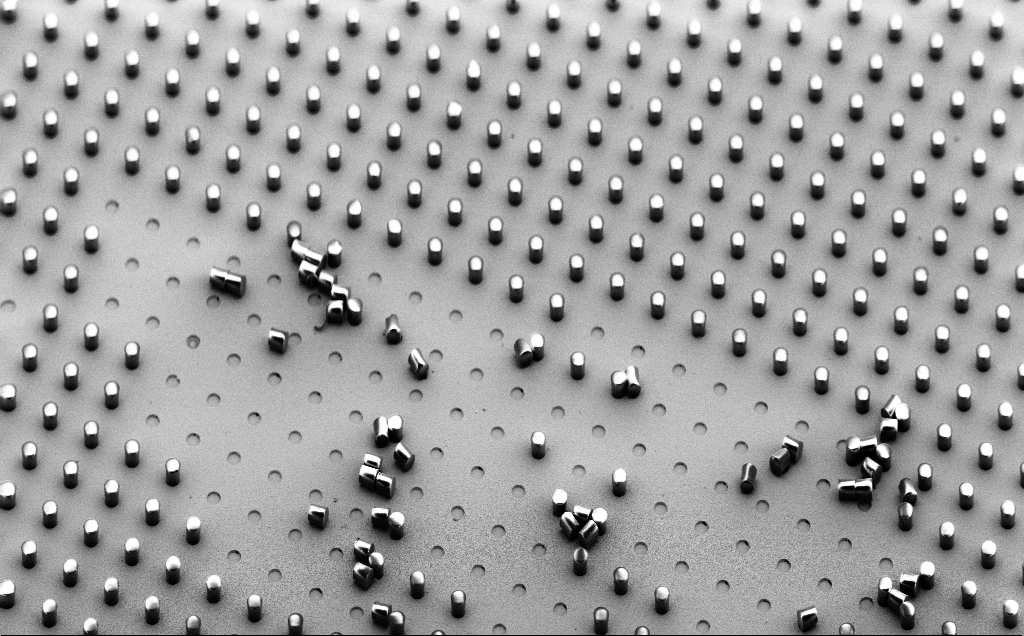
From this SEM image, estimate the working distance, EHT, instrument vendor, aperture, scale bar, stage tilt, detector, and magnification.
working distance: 8 mm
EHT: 5 kV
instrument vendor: Zeiss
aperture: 30 µm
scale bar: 200000 nm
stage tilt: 45°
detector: SE2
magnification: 0.279 K X